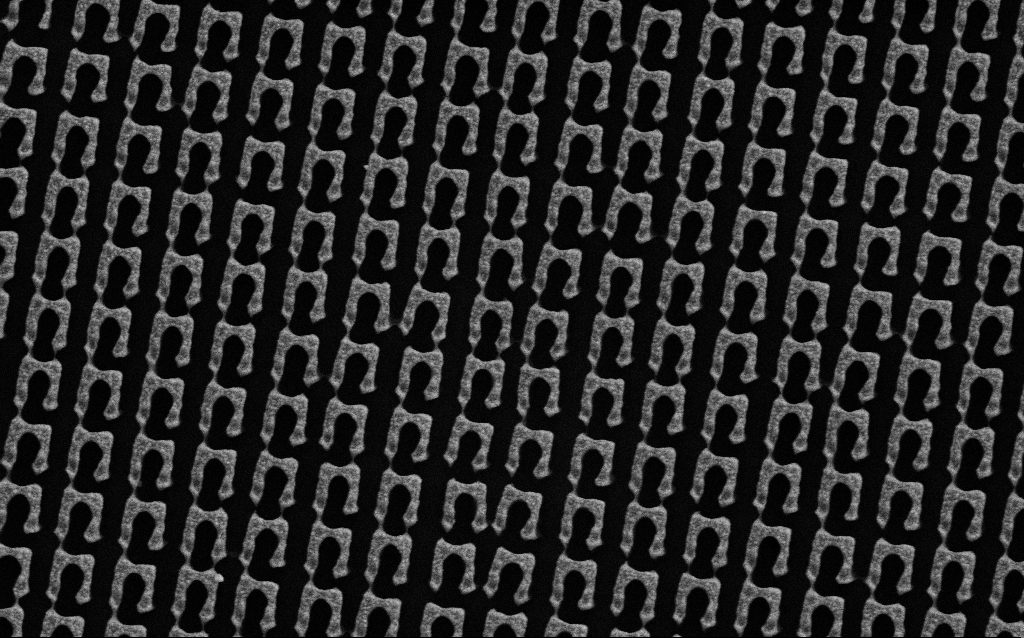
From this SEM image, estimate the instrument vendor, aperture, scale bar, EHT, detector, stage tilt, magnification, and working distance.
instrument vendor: Zeiss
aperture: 30 µm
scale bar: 1000 nm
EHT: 3 kV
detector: SE2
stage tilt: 0°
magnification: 49.73 K X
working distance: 4.6 mm